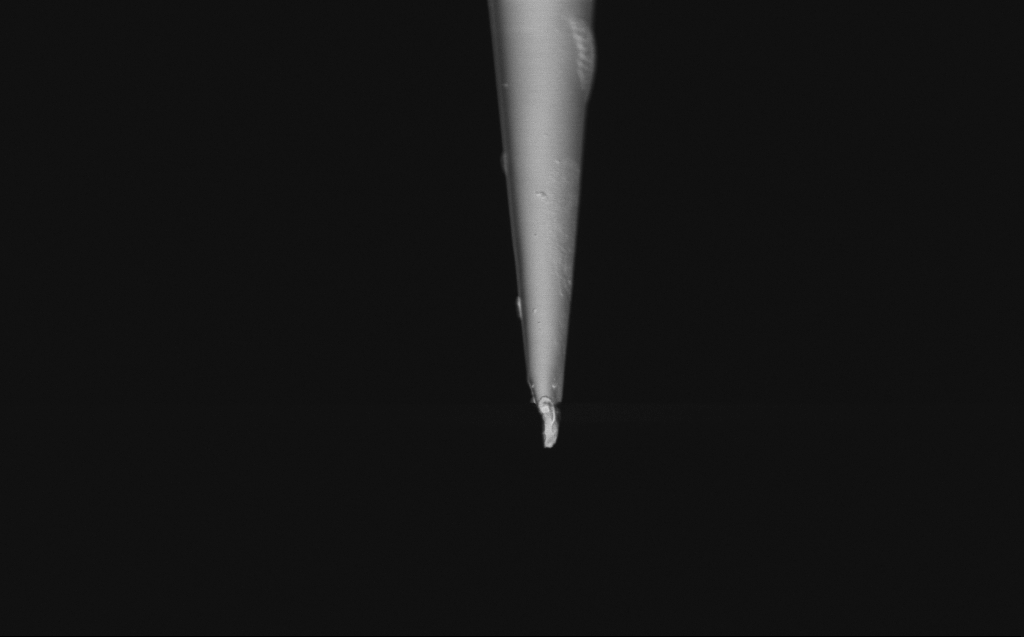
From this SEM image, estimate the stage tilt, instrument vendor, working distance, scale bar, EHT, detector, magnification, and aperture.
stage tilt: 45°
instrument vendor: Zeiss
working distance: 5 mm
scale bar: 2000 nm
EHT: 1 kV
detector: InLens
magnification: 10 K X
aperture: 30 µm